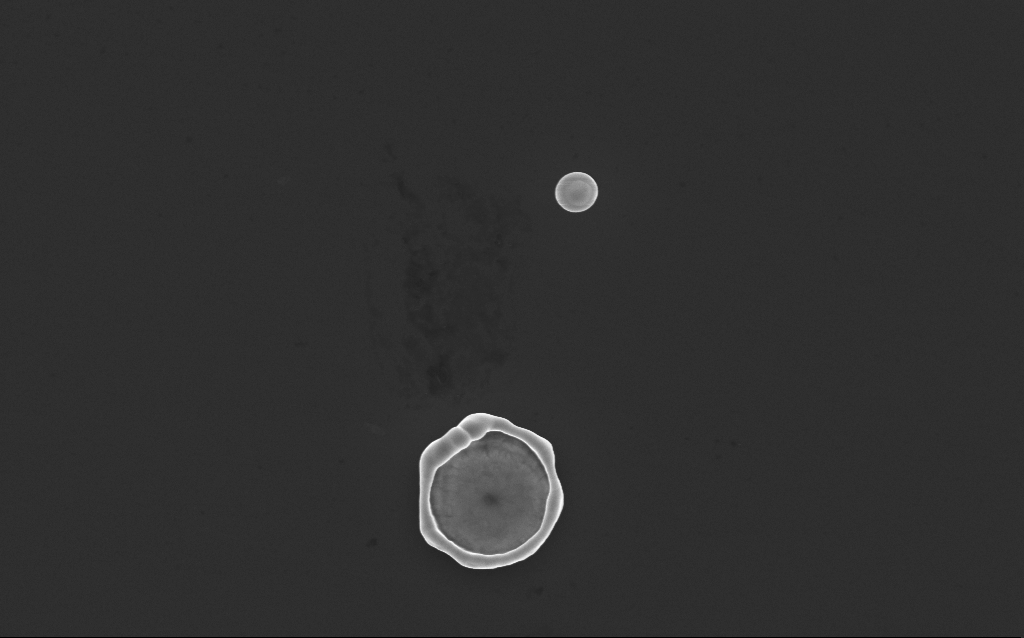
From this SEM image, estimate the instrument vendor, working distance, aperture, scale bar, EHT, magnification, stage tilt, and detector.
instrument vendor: Zeiss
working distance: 4 mm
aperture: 30 µm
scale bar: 2000 nm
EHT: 10 kV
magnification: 19.65 K X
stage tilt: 0°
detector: InLens